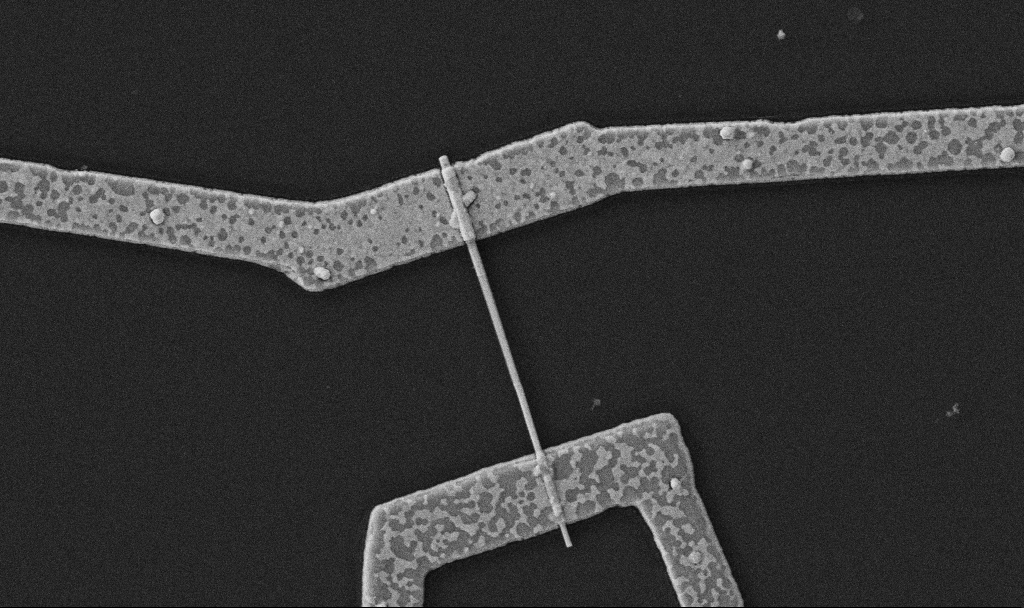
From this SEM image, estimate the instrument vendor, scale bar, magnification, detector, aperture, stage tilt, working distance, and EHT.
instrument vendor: Zeiss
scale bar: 1000 nm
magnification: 30 K X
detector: SE2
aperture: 30 µm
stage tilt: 0°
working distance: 10.7 mm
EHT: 5 kV